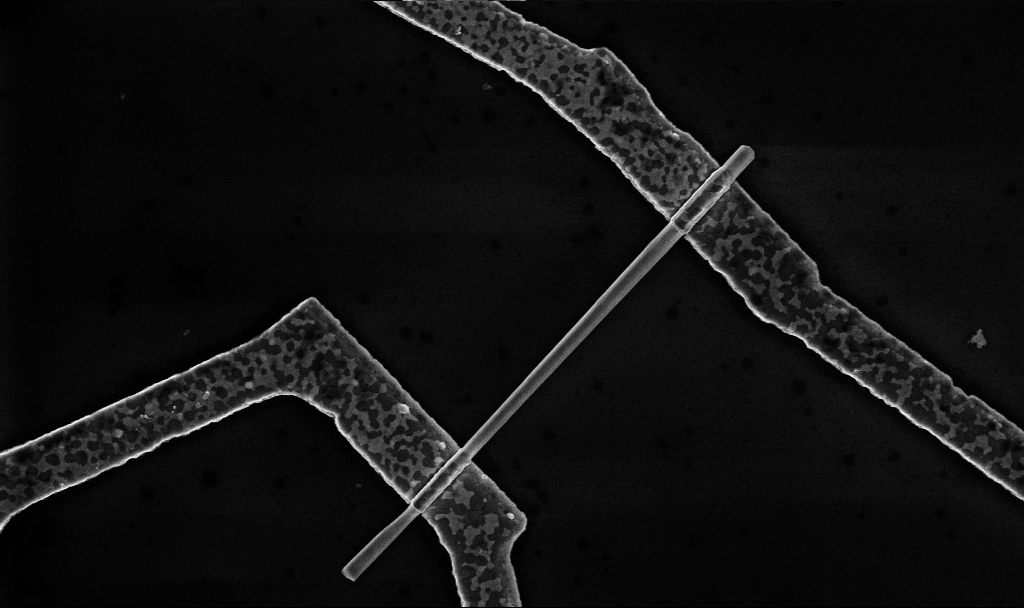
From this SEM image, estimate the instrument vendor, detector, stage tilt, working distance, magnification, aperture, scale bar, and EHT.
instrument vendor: Zeiss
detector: InLens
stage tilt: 0°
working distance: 8.7 mm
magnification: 30 K X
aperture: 30 µm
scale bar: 1000 nm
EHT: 5 kV